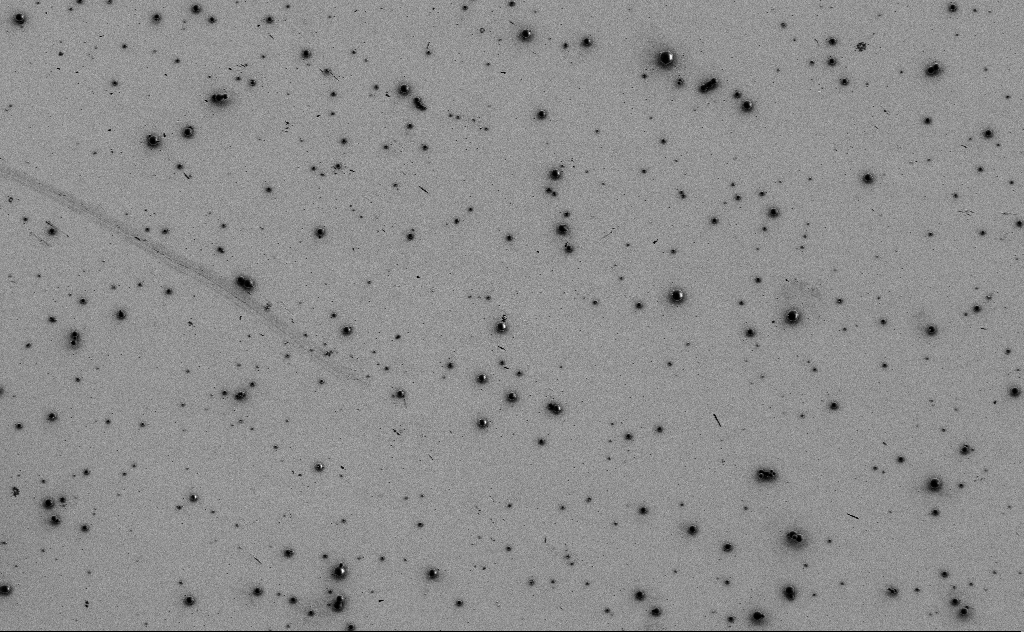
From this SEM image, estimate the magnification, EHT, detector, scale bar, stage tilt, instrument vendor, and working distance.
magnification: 1.65 K X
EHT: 3 kV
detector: SE2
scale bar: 10000 nm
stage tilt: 0°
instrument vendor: Zeiss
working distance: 10 mm